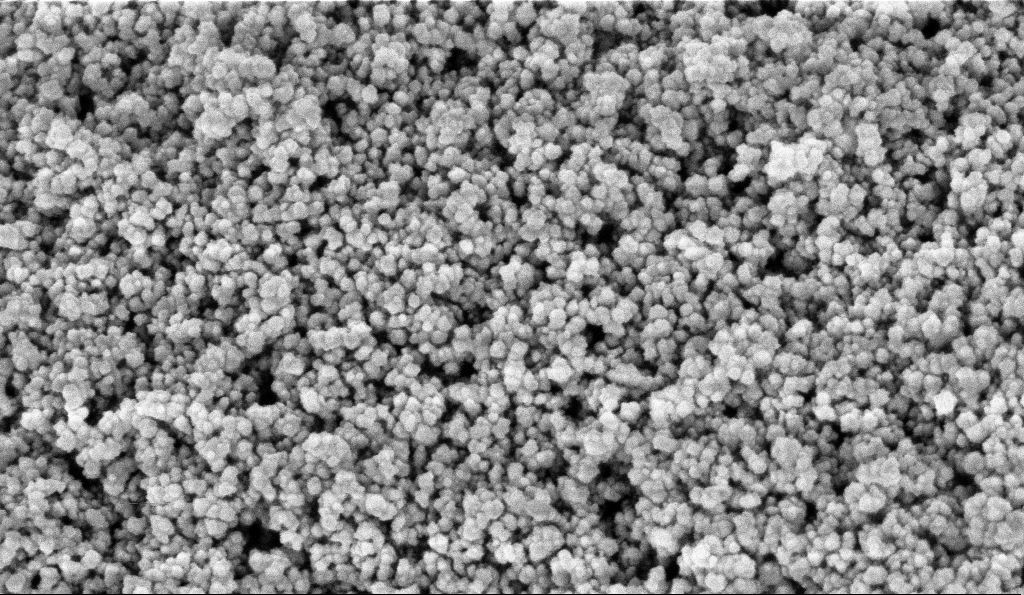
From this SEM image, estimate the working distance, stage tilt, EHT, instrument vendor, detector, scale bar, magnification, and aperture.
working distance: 5.9 mm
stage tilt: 0°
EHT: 5 kV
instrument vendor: Zeiss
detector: InLens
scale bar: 100 nm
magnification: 135 K X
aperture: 30 µm